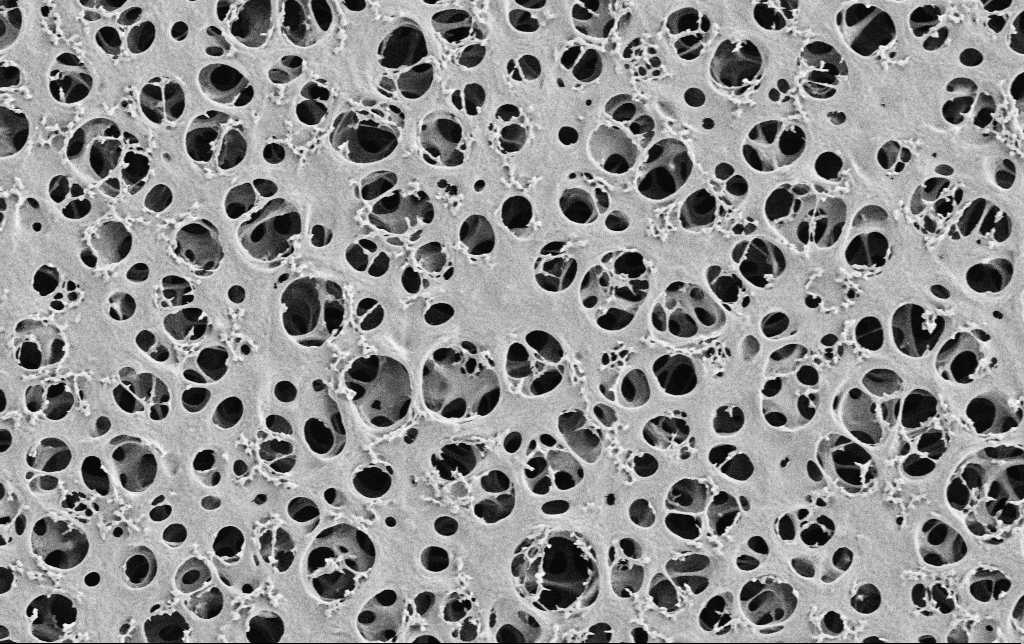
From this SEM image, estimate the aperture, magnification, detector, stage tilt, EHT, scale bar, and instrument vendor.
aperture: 30 µm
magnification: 10 K X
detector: SE2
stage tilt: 0°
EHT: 2 kV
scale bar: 2000 nm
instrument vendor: Zeiss